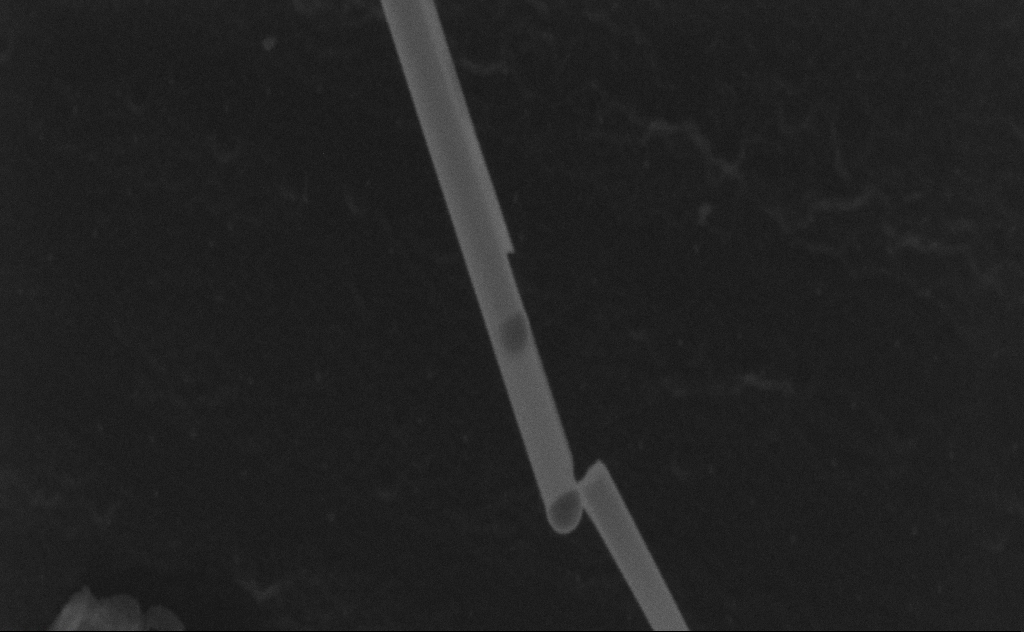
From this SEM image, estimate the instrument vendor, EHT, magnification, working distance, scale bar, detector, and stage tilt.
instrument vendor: Zeiss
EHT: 20 kV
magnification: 212.56 K X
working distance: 8 mm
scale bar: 200 nm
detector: SE2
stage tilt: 0°